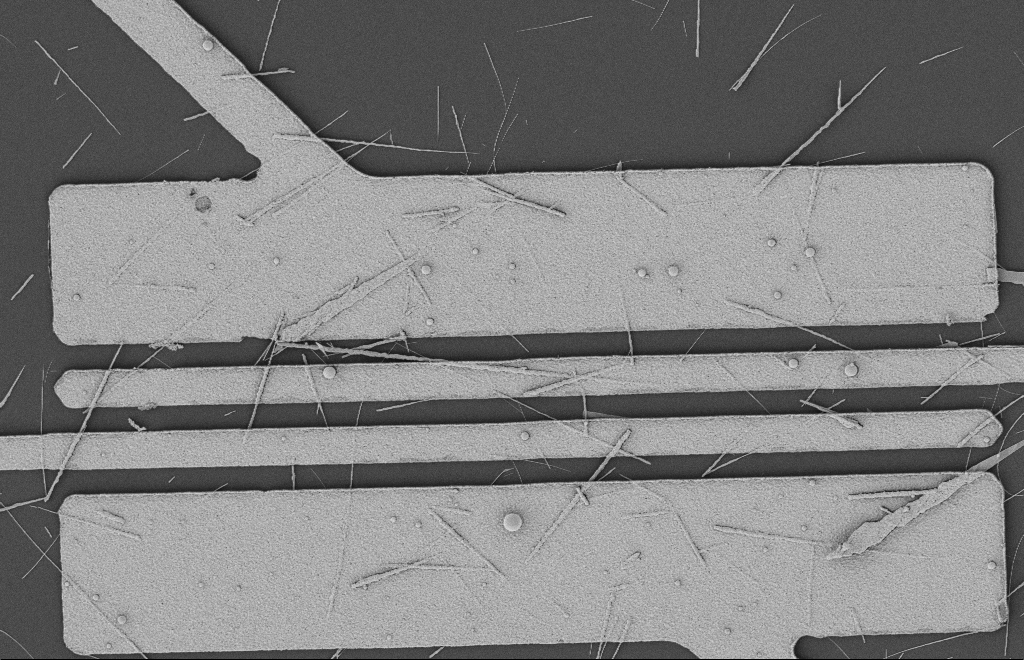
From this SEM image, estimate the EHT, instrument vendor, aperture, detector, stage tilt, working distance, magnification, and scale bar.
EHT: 2 kV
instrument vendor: Zeiss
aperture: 20 µm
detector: SE2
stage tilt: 0°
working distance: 12 mm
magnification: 5.65 K X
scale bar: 2000 nm